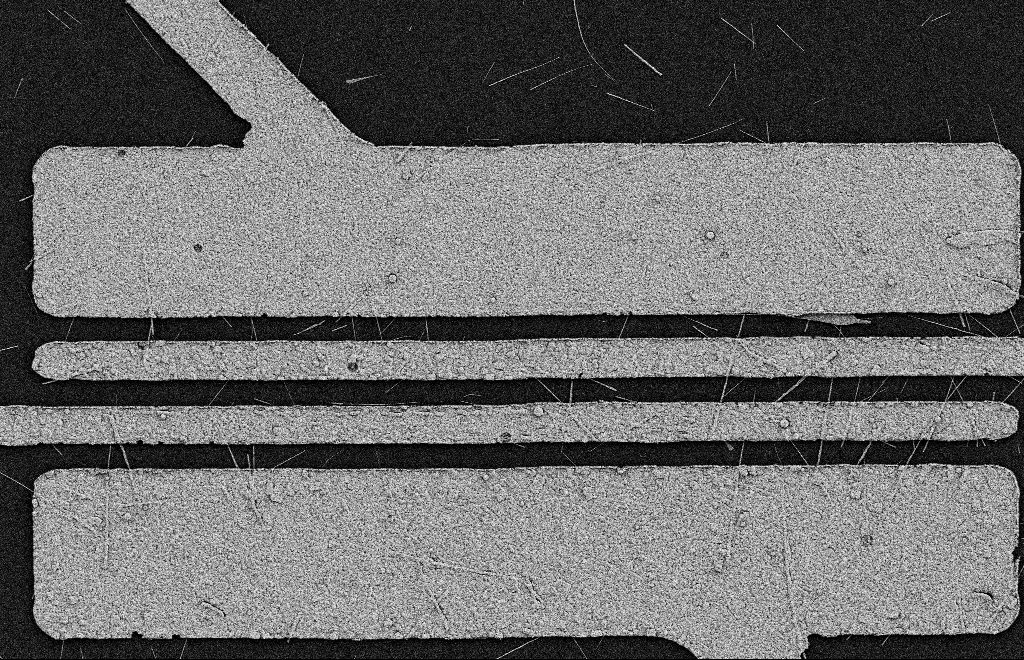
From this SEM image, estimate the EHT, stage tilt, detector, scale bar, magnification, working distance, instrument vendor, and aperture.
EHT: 2 kV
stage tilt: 0°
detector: SE2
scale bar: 2000 nm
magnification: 5.88 K X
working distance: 11 mm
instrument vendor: Zeiss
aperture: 20 µm